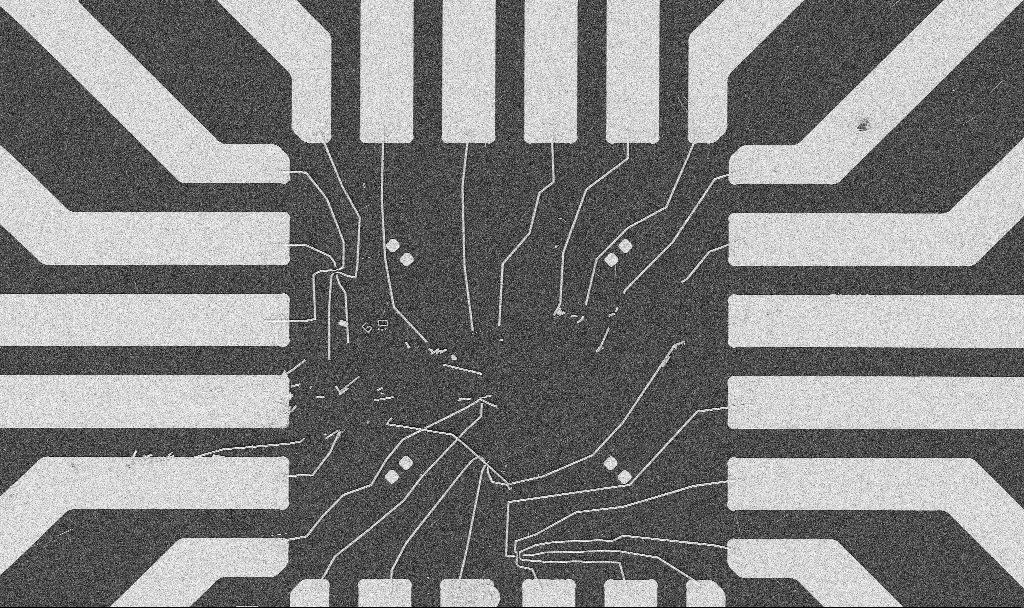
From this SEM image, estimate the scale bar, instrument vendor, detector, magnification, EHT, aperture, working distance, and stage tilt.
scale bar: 20000 nm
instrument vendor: Zeiss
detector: SE2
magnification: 1 K X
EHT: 5 kV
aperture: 30 µm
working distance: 10.7 mm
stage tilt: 0°